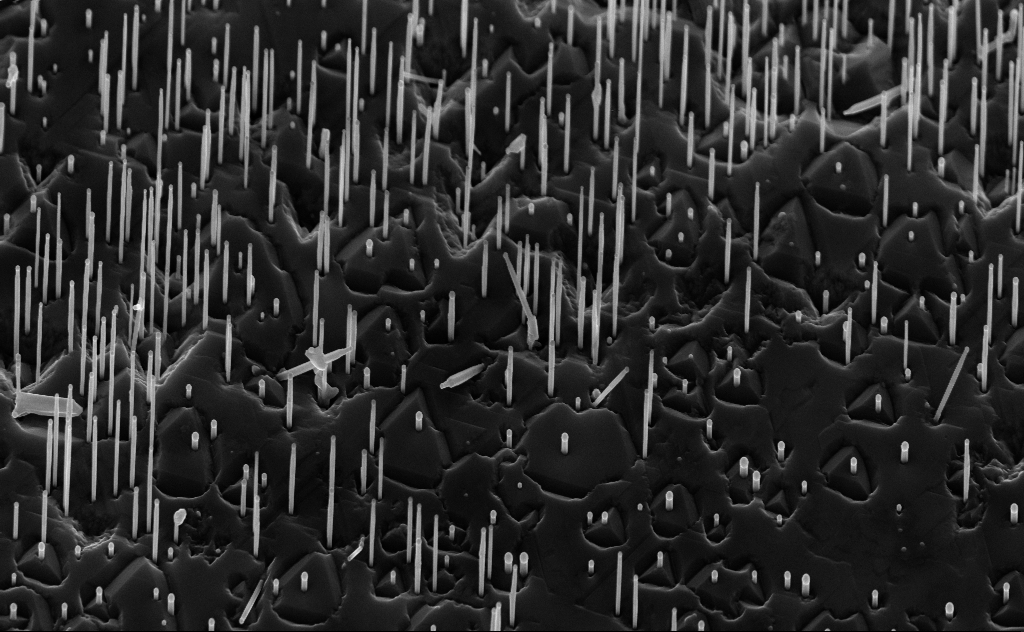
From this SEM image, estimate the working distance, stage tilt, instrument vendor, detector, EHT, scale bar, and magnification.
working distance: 6 mm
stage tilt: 45°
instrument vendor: Zeiss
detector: InLens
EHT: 10 kV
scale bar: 1000 nm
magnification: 20 K X